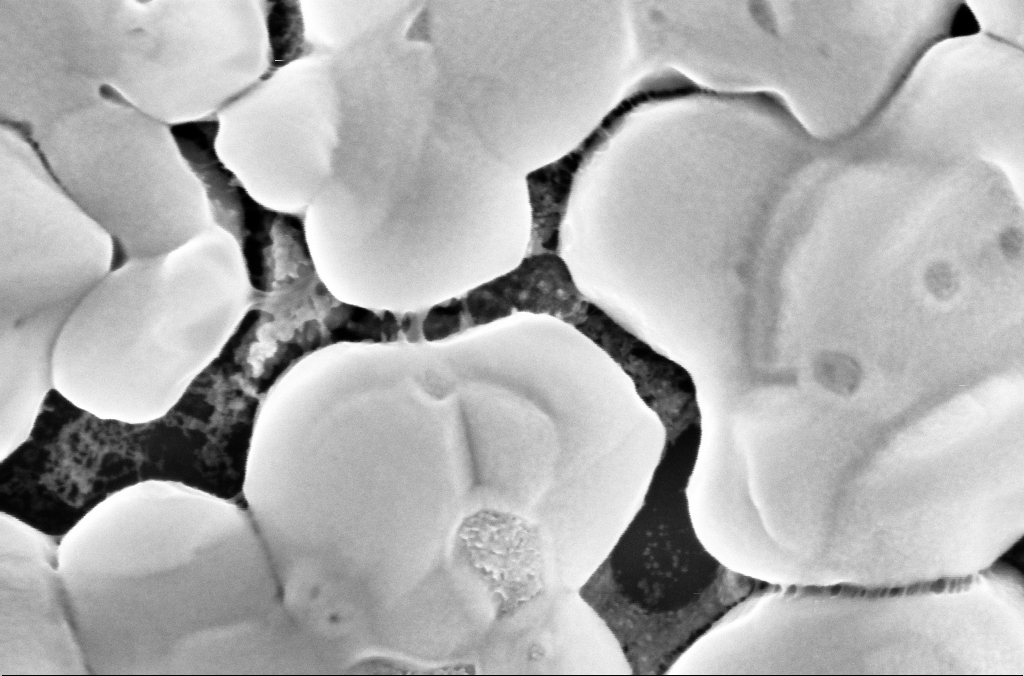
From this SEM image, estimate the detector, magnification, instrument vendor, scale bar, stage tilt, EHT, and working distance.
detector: InLens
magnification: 200 K X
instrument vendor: Zeiss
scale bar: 200 nm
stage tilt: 0°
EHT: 5 kV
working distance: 3.1 mm